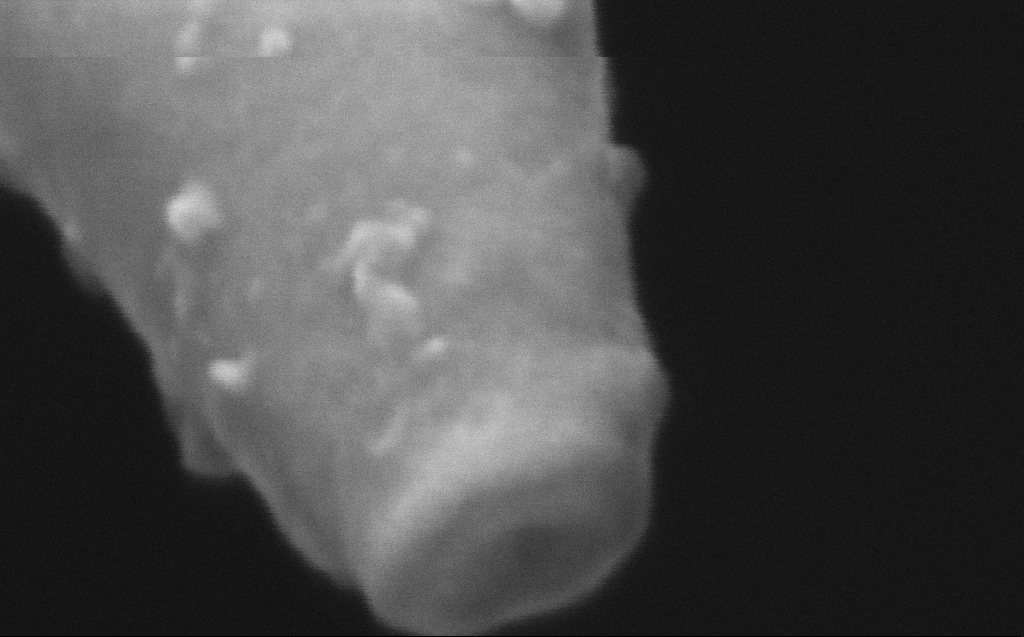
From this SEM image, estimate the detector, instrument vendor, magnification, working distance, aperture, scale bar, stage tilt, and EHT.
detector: InLens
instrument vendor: Zeiss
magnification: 1000 K X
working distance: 6 mm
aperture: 30 µm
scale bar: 20 nm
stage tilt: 45°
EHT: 5 kV